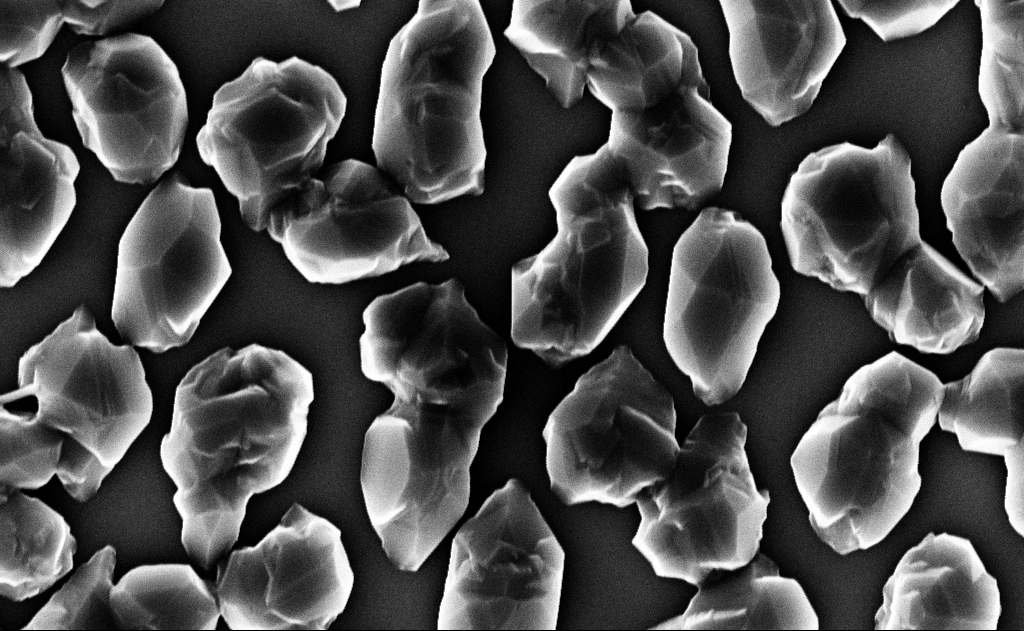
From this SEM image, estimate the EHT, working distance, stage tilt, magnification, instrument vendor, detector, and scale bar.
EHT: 10 kV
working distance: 12 mm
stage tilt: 0°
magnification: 50 K X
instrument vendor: Zeiss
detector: InLens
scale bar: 1000 nm